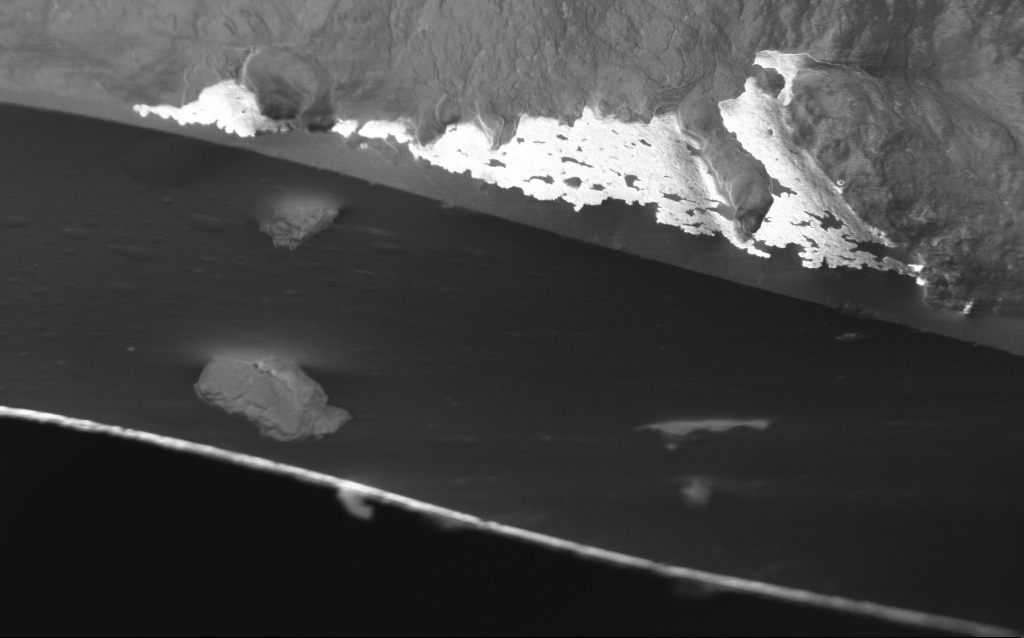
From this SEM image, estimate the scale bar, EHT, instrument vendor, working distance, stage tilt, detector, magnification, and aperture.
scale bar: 2000 nm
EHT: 1 kV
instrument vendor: Zeiss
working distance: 5 mm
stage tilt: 45°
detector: InLens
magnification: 14.9 K X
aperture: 30 µm